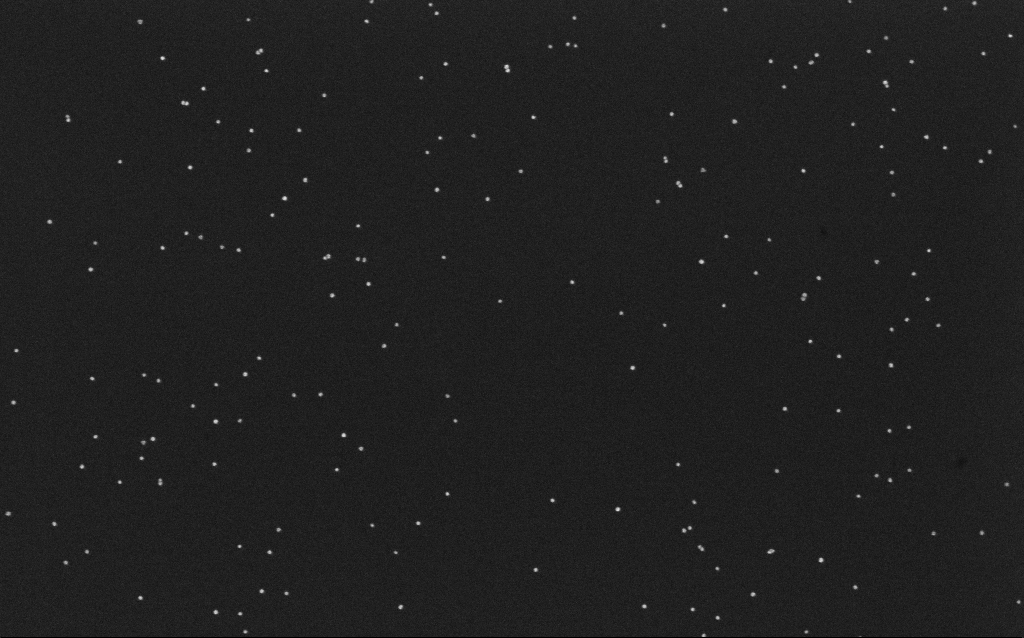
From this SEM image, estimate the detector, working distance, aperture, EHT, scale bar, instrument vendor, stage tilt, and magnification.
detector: InLens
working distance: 6.6 mm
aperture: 30 µm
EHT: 10 kV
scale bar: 200 nm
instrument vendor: Zeiss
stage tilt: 0°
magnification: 100 K X